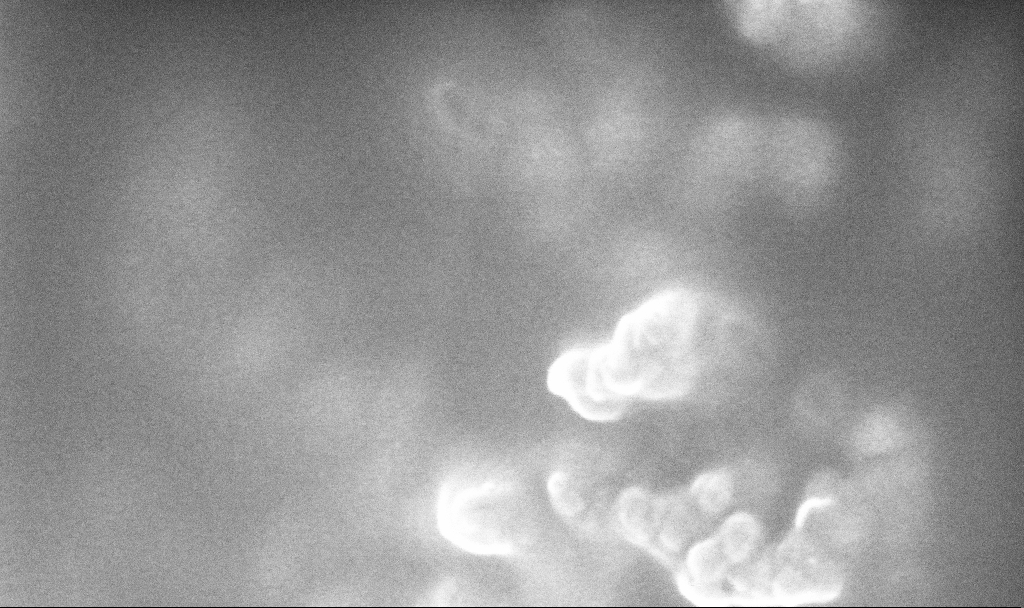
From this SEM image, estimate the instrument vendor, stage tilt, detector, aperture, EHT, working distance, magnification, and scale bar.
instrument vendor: Zeiss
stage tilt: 0°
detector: InLens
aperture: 30 µm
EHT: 10 kV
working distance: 2.4 mm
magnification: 344.61 K X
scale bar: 200 nm